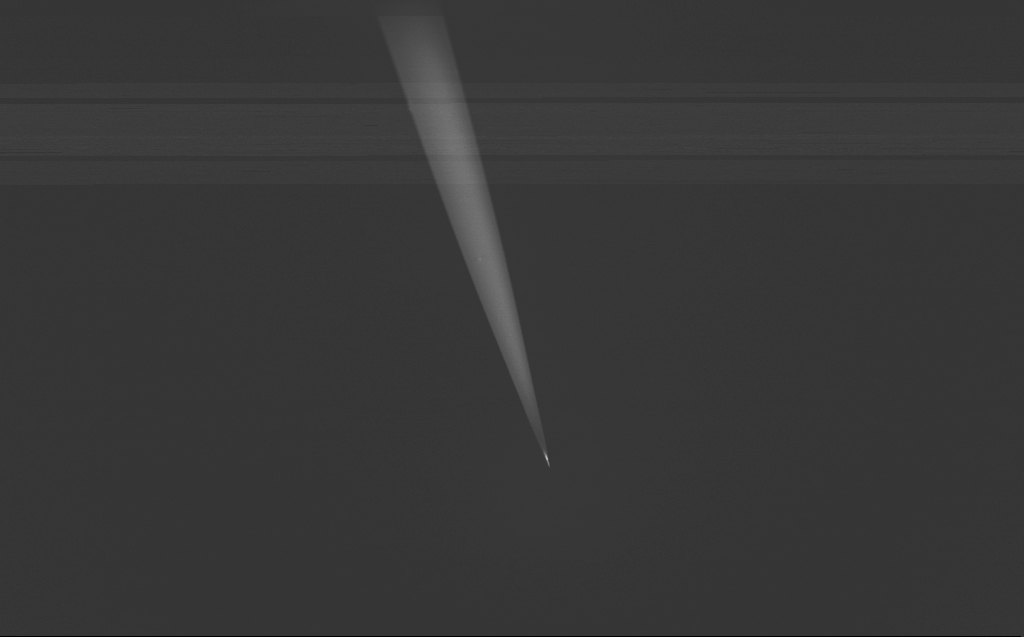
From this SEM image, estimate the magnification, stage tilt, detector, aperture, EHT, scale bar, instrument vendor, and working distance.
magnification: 1 K X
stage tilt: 39.3°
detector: InLens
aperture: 30 µm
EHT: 0.8 kV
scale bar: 20000 nm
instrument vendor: Zeiss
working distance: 5 mm